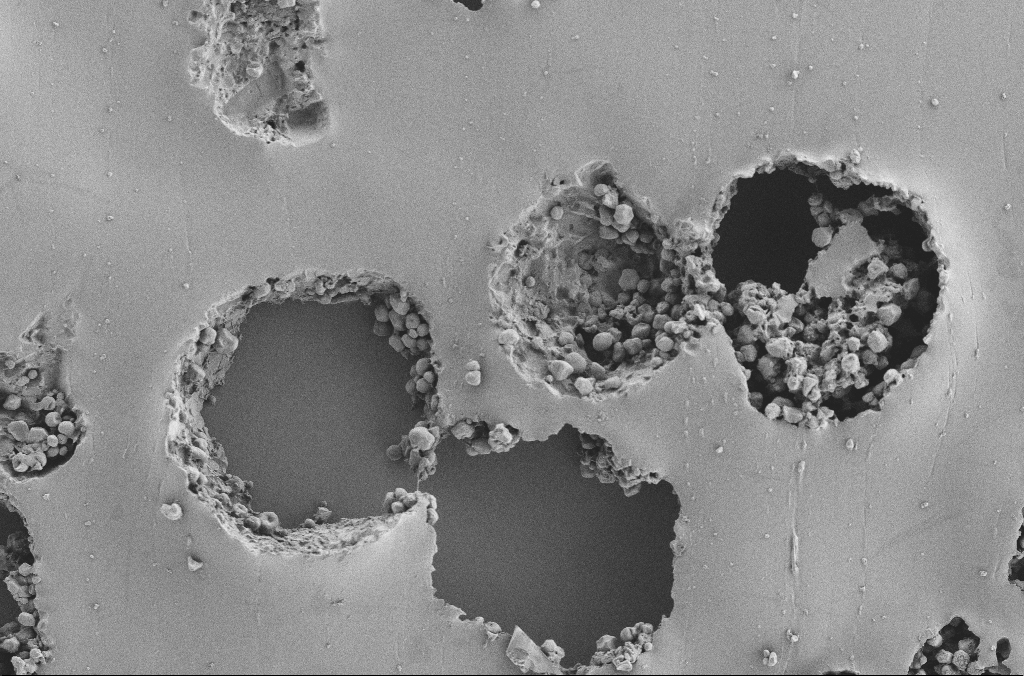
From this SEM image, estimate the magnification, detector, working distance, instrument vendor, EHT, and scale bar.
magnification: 0.5 K X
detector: SE2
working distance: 3.7 mm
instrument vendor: Zeiss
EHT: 2 kV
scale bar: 100000 nm